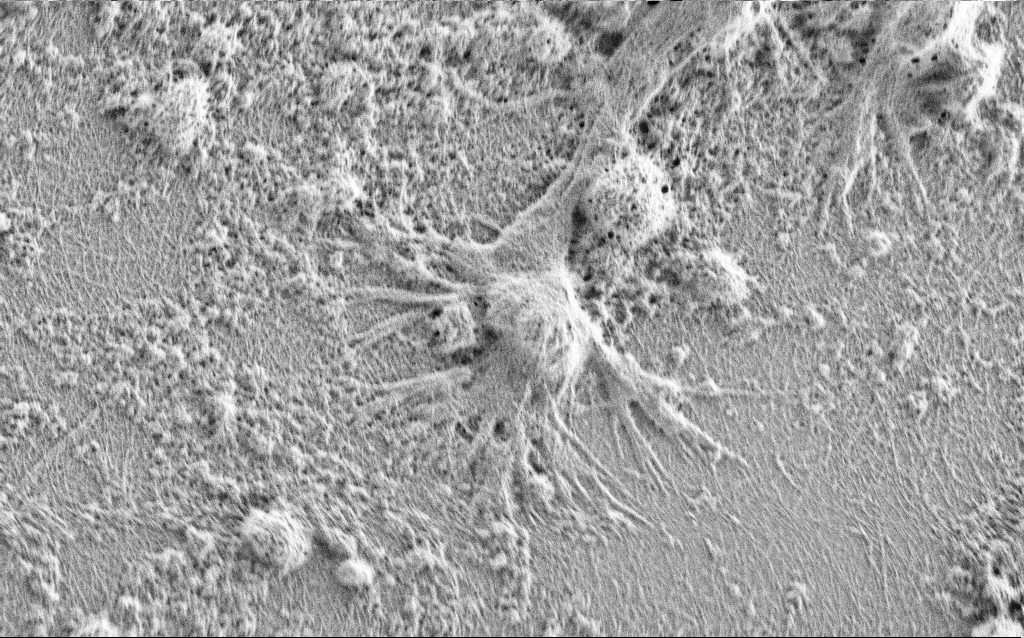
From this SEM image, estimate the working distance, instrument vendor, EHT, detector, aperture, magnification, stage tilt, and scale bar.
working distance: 3 mm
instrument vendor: Zeiss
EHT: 0.9 kV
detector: SE2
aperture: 30 µm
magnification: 10 K X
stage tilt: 0°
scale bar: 2000 nm